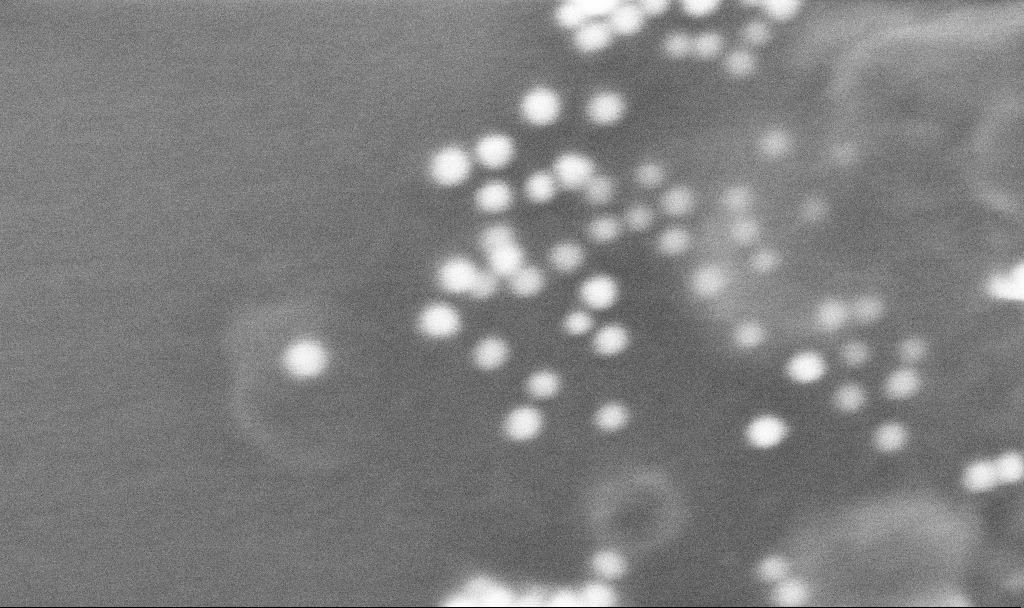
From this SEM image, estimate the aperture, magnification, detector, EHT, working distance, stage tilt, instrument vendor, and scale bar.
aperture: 30 µm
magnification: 564.05 K X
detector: InLens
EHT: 10 kV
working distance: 3.4 mm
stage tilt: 0°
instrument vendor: Zeiss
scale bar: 100 nm